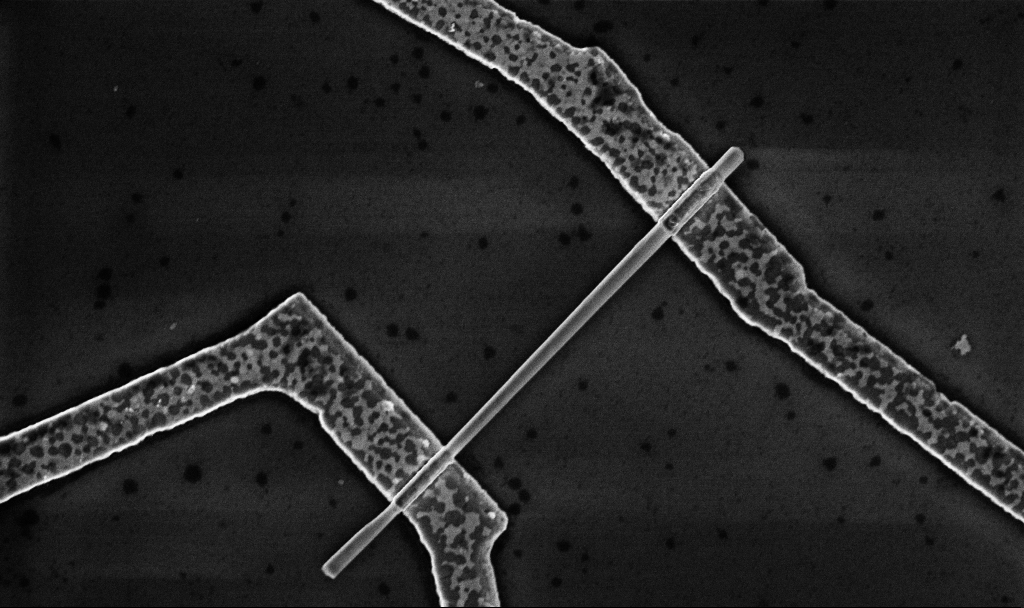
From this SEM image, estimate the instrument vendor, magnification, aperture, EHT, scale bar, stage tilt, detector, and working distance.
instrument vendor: Zeiss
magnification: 30 K X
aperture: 30 µm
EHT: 5 kV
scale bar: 1000 nm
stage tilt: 0°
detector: InLens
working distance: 8.7 mm